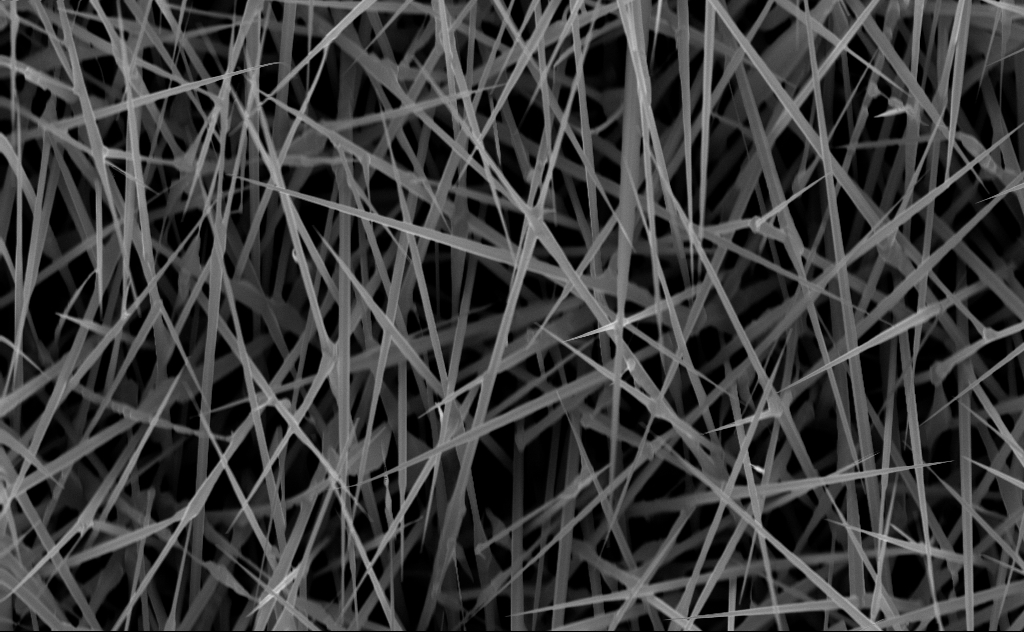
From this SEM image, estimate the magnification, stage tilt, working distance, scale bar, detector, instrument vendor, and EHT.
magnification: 20 K X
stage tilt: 0°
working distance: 5 mm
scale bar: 2000 nm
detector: InLens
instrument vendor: Zeiss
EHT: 10 kV